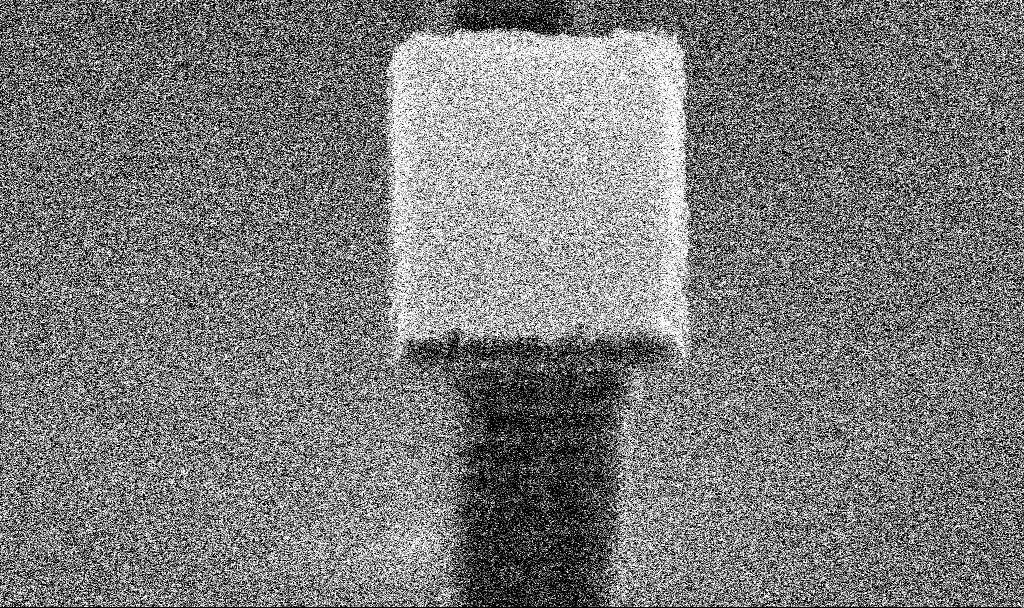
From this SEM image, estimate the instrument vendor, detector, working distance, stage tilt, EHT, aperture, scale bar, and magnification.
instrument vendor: Zeiss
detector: SE2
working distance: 6.5 mm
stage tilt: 45°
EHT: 5 kV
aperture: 30 µm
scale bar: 1000 nm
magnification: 37.87 K X